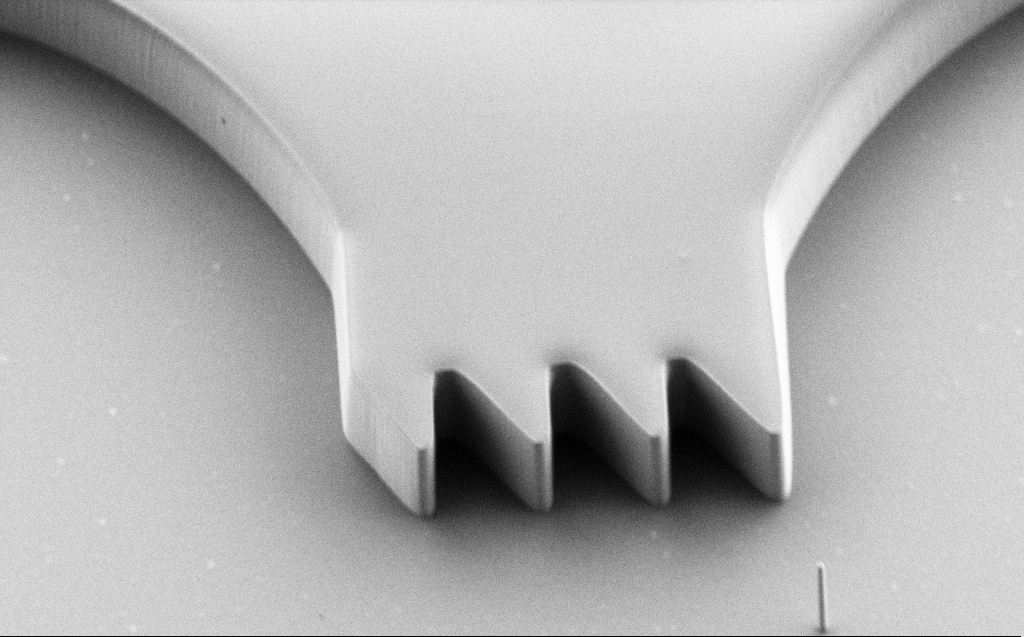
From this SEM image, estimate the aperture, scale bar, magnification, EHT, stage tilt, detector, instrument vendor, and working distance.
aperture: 30 µm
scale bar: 10000 nm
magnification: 3.75 K X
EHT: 1 kV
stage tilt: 30°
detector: SE2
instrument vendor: Zeiss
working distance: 6 mm